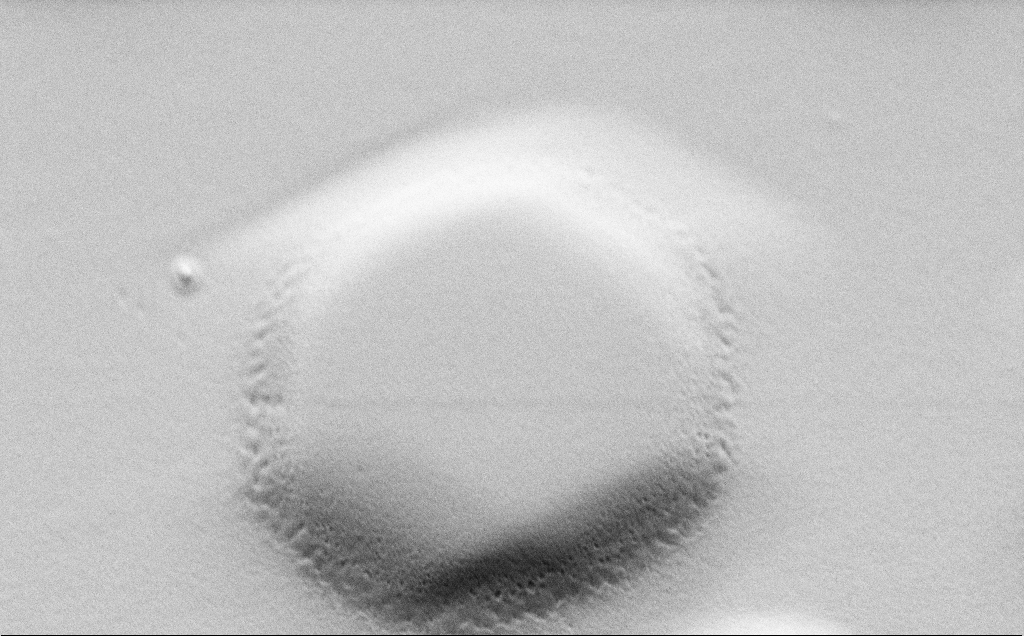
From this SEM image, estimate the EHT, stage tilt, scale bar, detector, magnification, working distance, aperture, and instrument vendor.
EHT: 1.5 kV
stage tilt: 45°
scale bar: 1000 nm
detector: SE2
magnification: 15.36 K X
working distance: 4 mm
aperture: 30 µm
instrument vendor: Zeiss